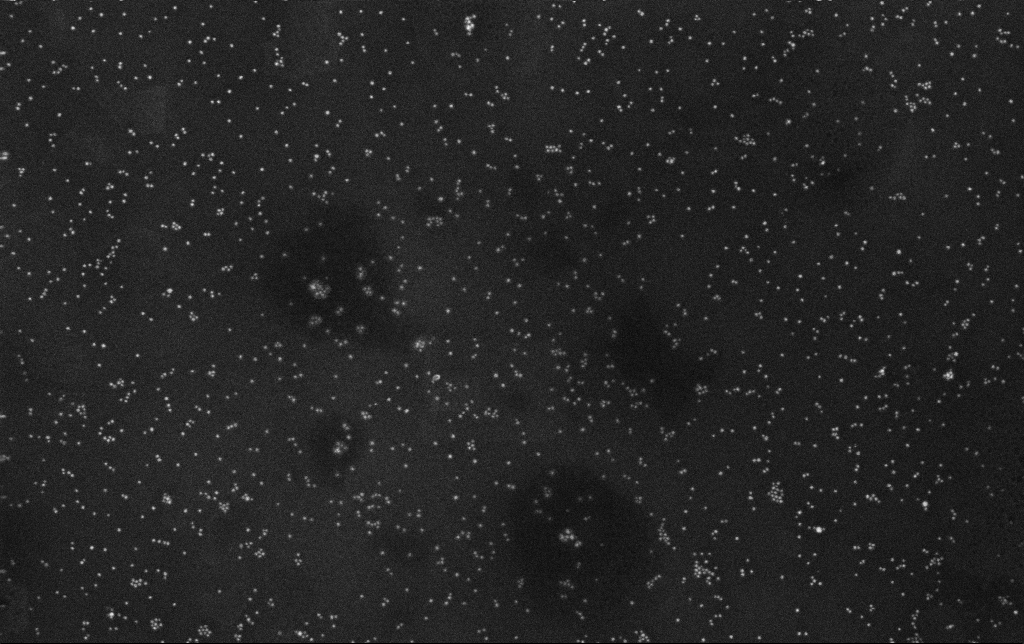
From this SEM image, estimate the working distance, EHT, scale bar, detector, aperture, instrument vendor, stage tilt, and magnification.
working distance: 3.4 mm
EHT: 10 kV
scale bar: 200 nm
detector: InLens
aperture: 30 µm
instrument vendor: Zeiss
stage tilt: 0°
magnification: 100 K X